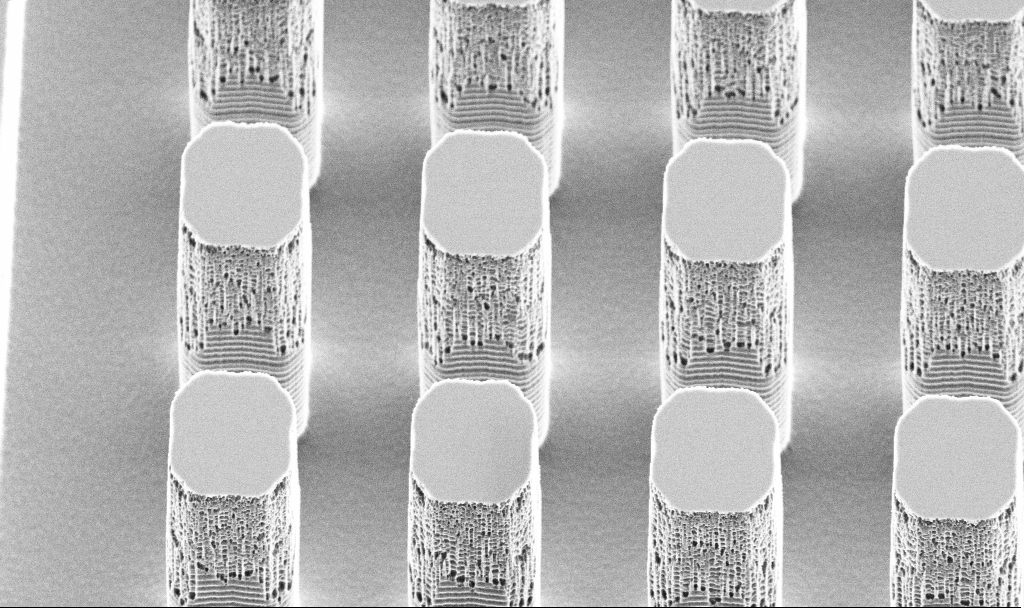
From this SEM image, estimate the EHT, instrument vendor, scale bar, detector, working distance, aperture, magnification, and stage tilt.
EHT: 5 kV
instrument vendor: Zeiss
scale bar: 10000 nm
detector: SE2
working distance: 16.6 mm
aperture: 30 µm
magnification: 4.42 K X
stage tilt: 45°